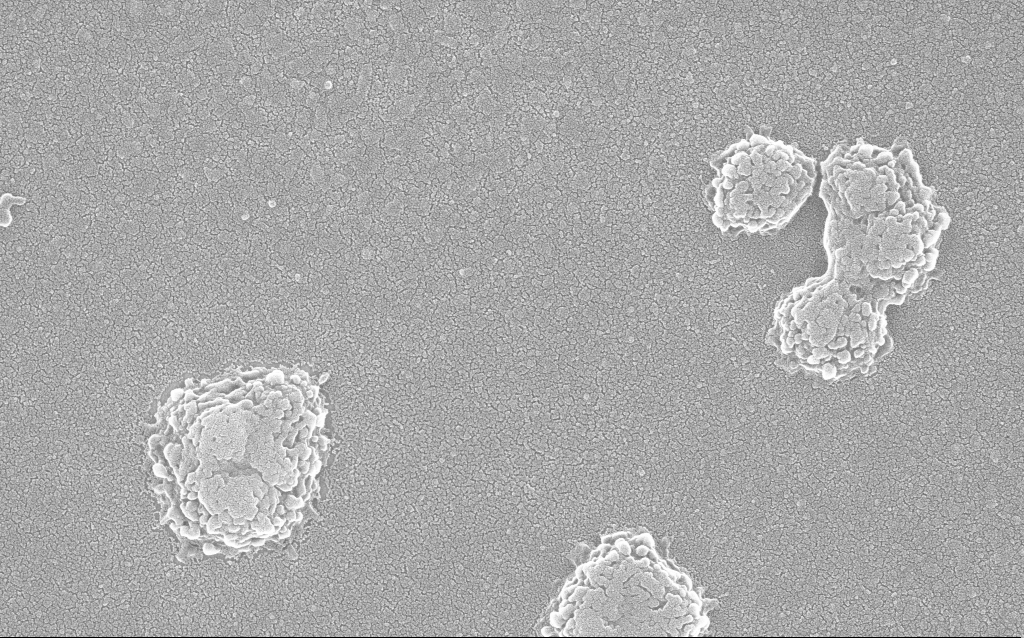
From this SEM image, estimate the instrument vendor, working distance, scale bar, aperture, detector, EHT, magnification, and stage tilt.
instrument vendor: Zeiss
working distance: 1.8 mm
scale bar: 200 nm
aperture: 30 µm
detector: InLens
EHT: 20 kV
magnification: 100 K X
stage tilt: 0°